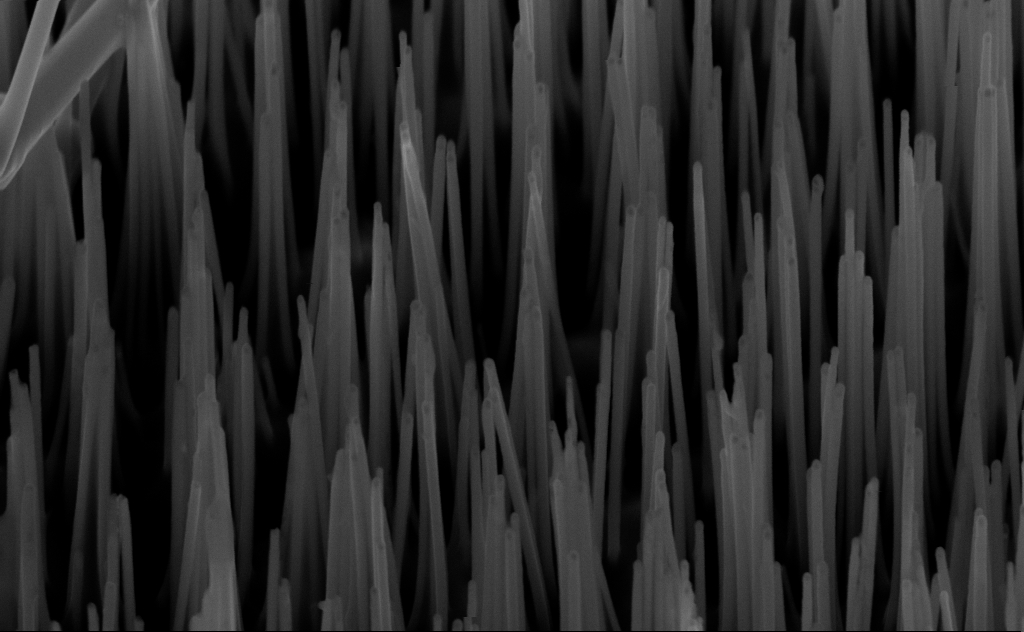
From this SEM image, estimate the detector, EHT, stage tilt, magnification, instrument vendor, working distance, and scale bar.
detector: InLens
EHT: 10 kV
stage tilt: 45°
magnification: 80 K X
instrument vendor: Zeiss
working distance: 6 mm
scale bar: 200 nm